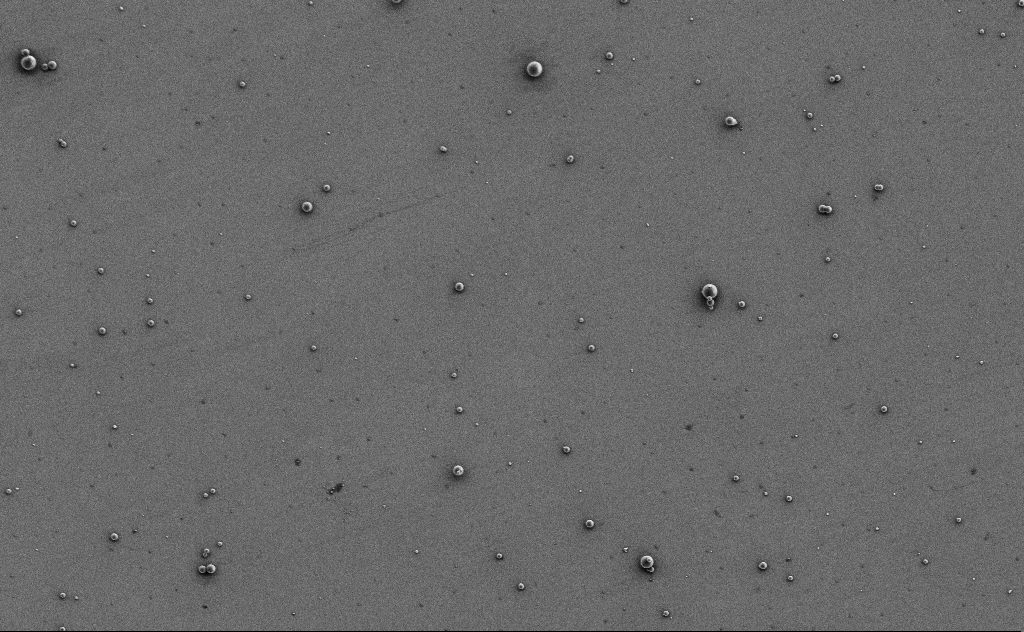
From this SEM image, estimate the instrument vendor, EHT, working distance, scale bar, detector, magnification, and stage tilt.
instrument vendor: Zeiss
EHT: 3 kV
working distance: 11 mm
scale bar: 10000 nm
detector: SE2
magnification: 2.24 K X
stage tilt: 0°